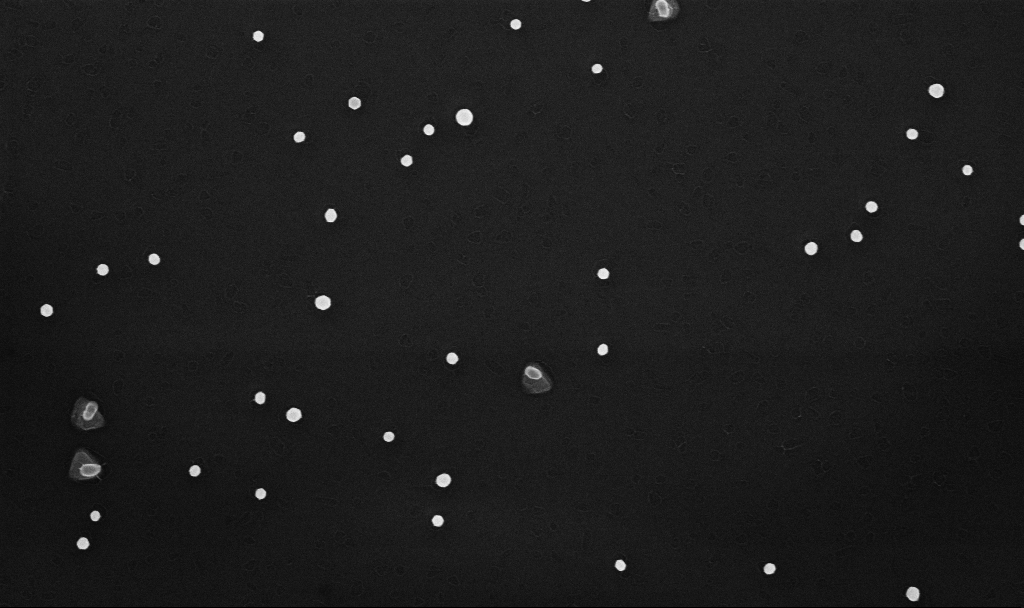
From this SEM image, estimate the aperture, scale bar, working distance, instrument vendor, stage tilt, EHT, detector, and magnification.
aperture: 30 µm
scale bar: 1000 nm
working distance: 3.3 mm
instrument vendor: Zeiss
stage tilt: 0°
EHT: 10 kV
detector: InLens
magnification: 70 K X